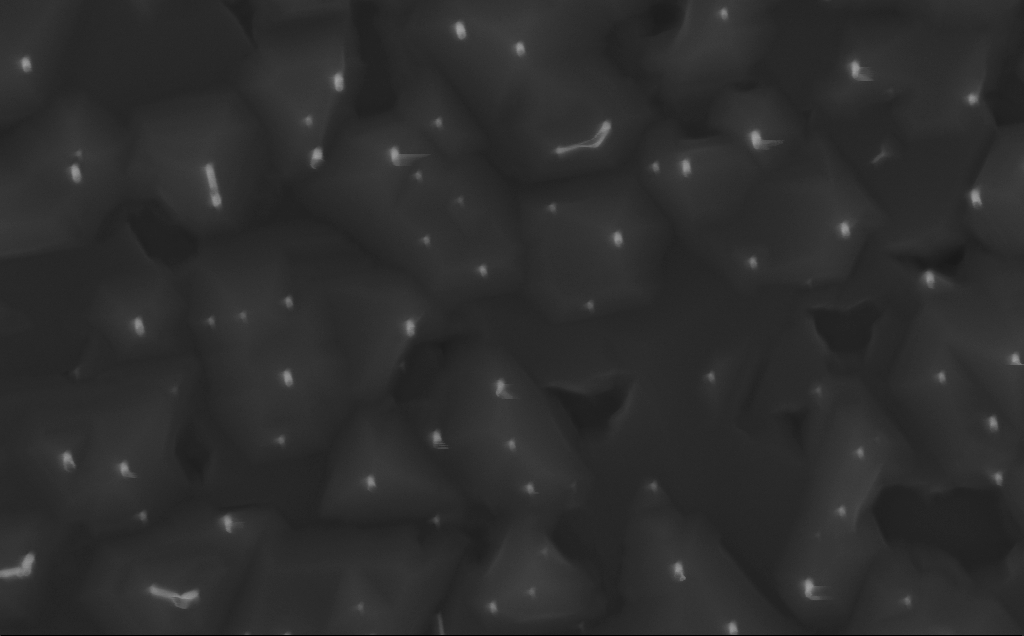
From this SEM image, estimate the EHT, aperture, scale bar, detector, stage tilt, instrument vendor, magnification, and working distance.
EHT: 10 kV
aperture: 30 µm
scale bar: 200 nm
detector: InLens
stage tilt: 0°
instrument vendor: Zeiss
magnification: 80 K X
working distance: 3 mm